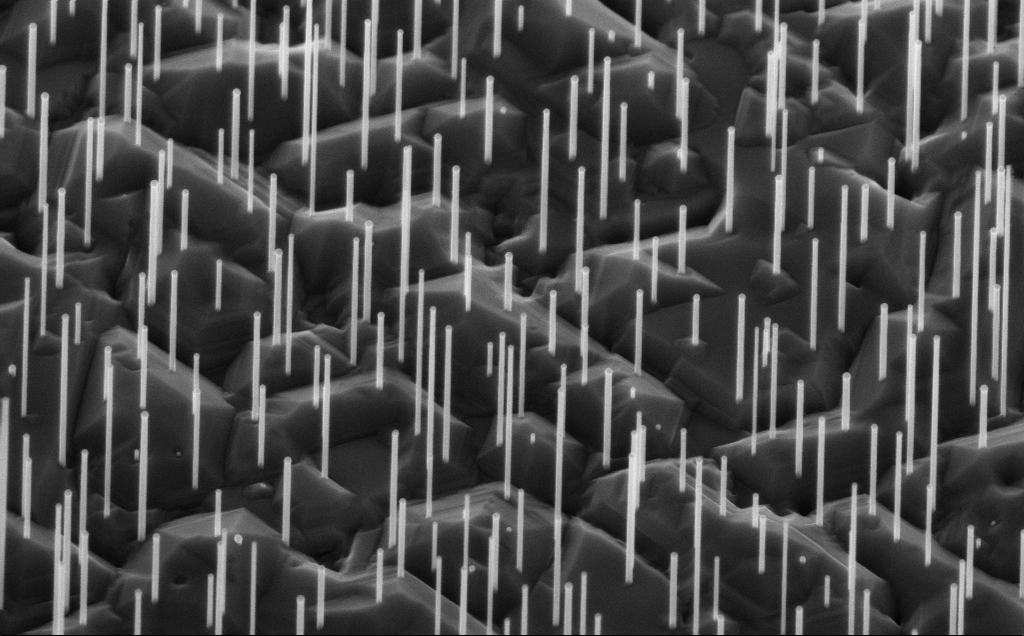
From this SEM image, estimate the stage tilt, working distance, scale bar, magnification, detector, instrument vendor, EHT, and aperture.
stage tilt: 45°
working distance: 6 mm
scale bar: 1000 nm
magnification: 40 K X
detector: InLens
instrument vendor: Zeiss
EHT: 10 kV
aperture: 30 µm